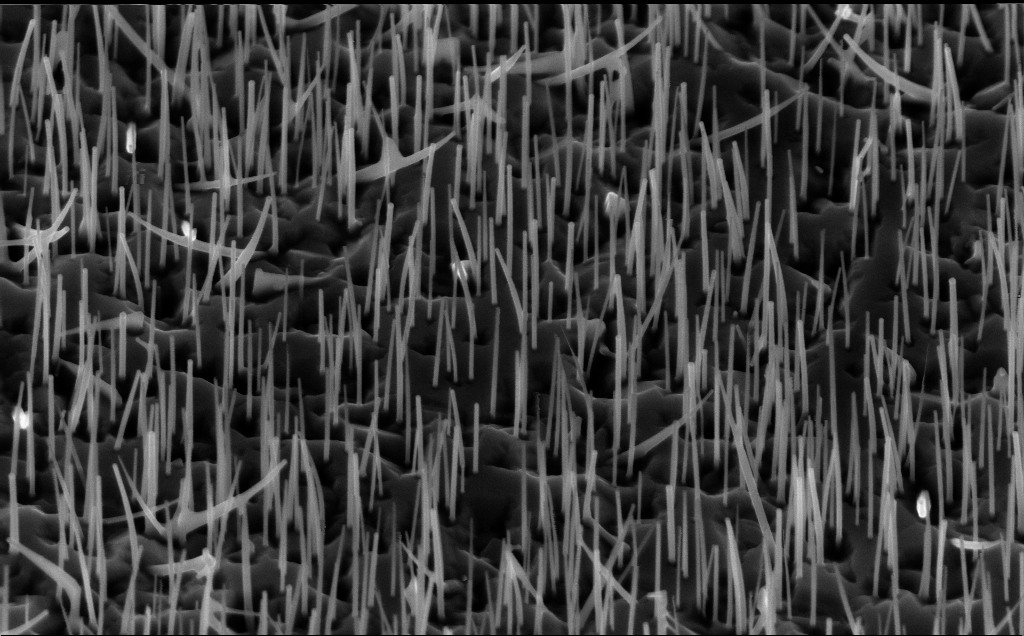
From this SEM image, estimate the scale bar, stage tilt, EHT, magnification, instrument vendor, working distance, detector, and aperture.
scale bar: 1000 nm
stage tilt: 45°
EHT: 10 kV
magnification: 40 K X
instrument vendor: Zeiss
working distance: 5 mm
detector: InLens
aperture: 30 µm